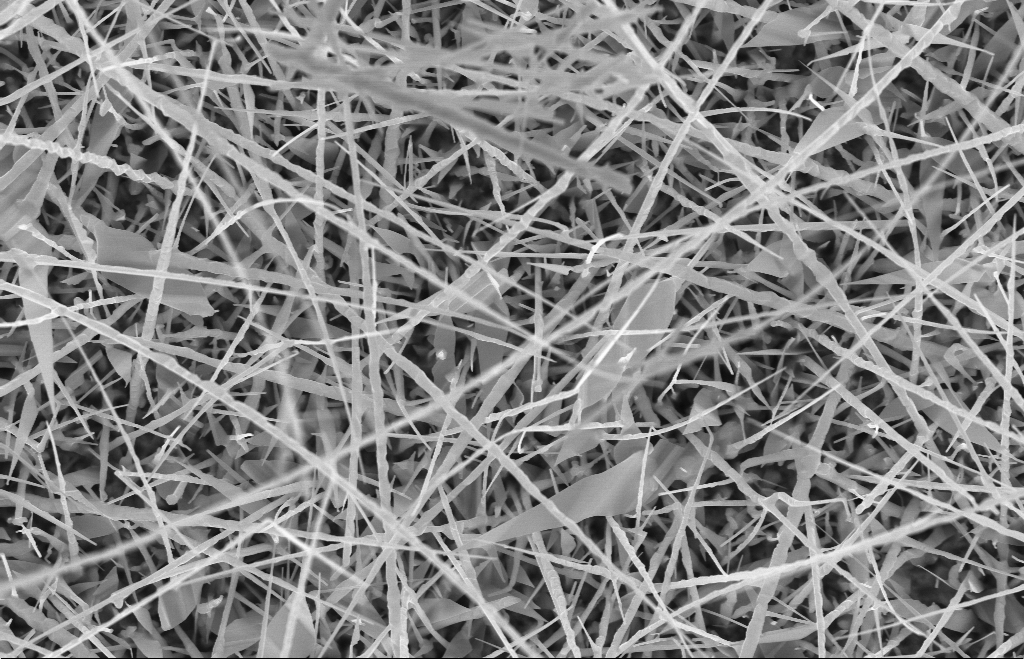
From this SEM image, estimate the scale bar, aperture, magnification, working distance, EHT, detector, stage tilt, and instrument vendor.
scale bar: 1000 nm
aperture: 30 µm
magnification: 20 K X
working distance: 9 mm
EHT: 10 kV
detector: InLens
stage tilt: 0°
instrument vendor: Zeiss